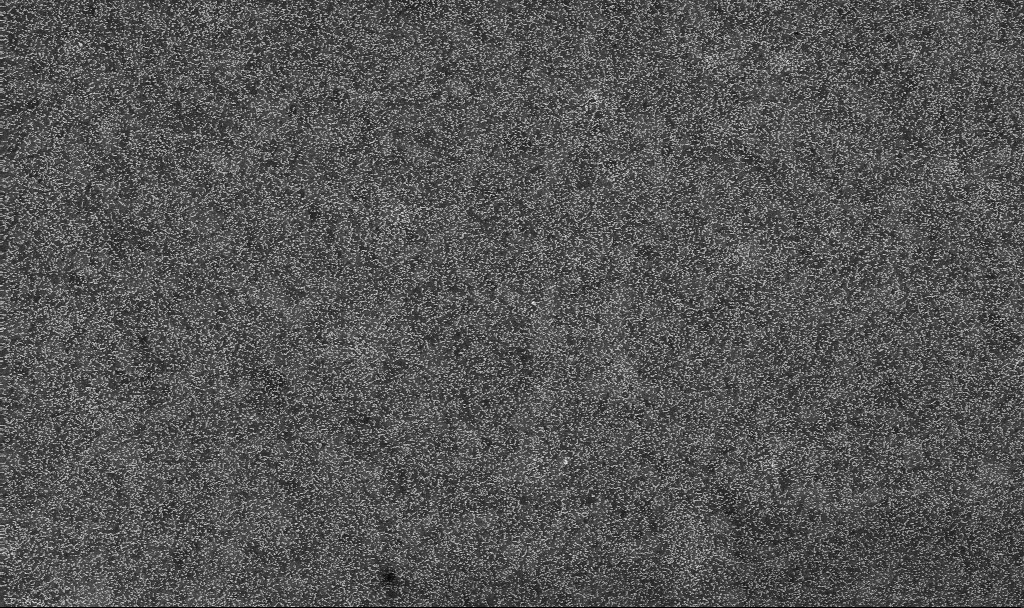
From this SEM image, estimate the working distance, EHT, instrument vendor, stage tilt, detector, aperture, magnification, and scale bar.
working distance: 3.3 mm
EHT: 10 kV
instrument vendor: Zeiss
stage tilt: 0°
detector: InLens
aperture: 30 µm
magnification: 20 K X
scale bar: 1000 nm